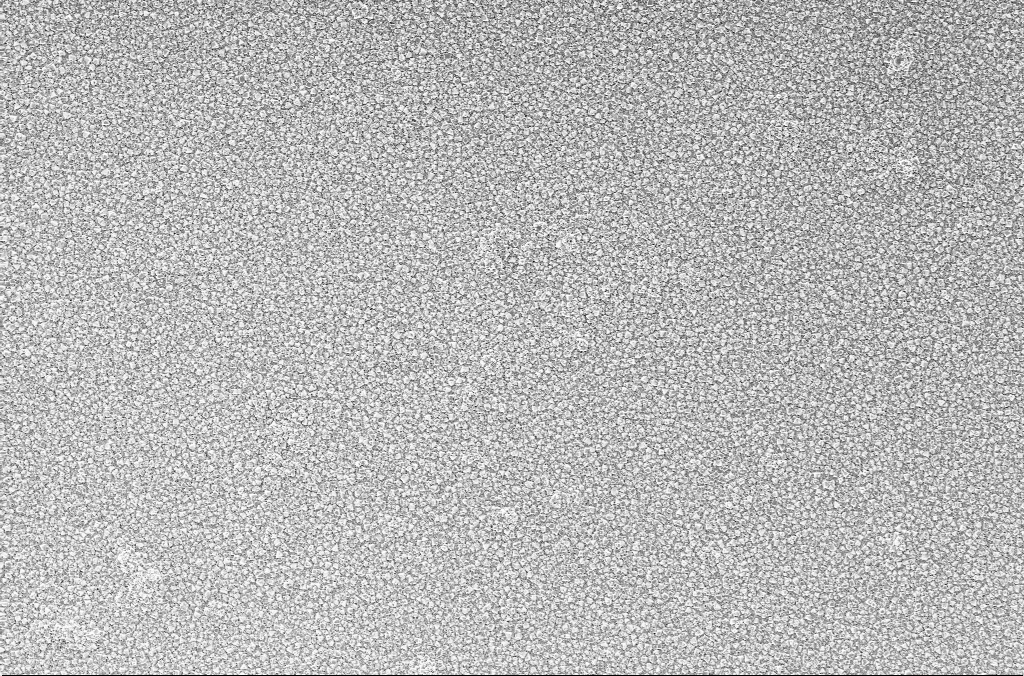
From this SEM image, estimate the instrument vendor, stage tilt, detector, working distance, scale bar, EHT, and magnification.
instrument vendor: Zeiss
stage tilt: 0°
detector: InLens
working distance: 1.9 mm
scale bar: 10000 nm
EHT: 2 kV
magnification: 5 K X